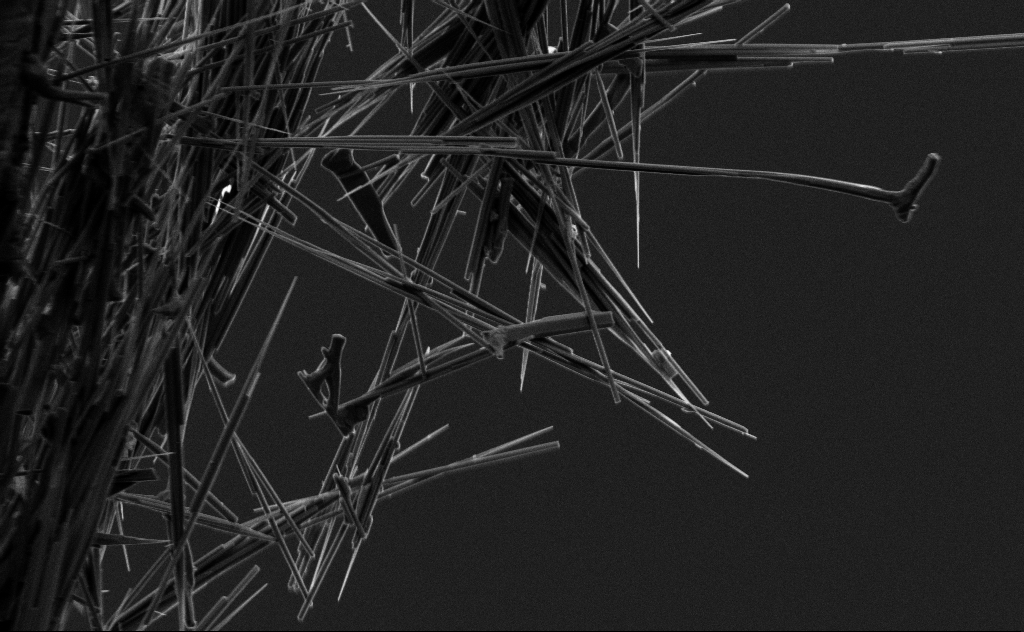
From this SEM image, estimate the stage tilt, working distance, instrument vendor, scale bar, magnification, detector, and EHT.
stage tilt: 0°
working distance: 10 mm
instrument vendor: Zeiss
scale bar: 2000 nm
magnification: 13.77 K X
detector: SE2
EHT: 3 kV